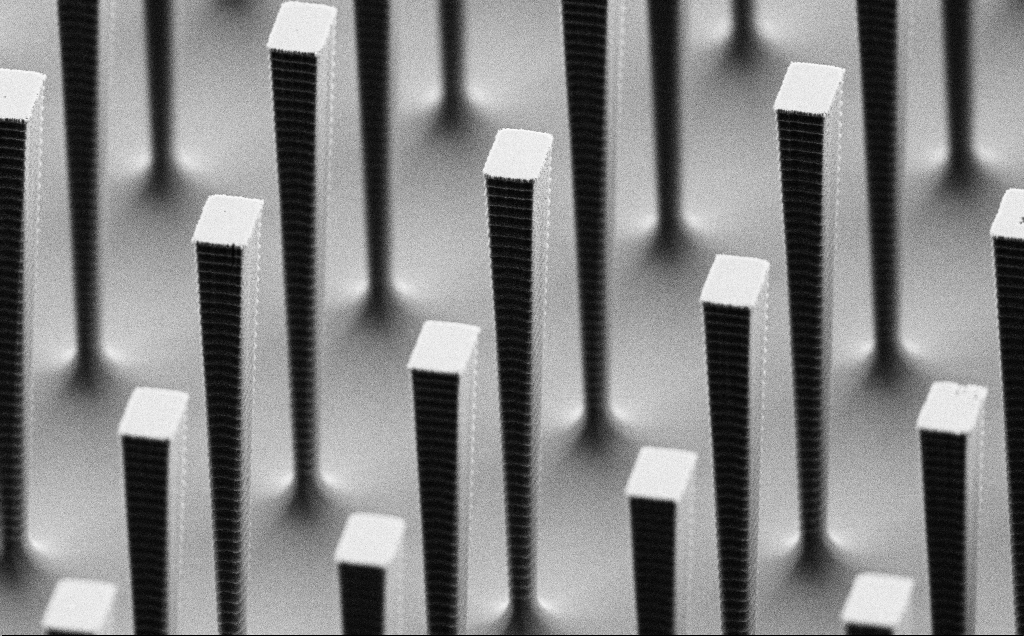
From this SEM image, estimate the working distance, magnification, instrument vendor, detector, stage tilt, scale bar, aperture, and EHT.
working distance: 5.4 mm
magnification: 10.54 K X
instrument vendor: Zeiss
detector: SE2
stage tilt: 60°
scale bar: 2000 nm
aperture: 30 µm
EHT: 3 kV